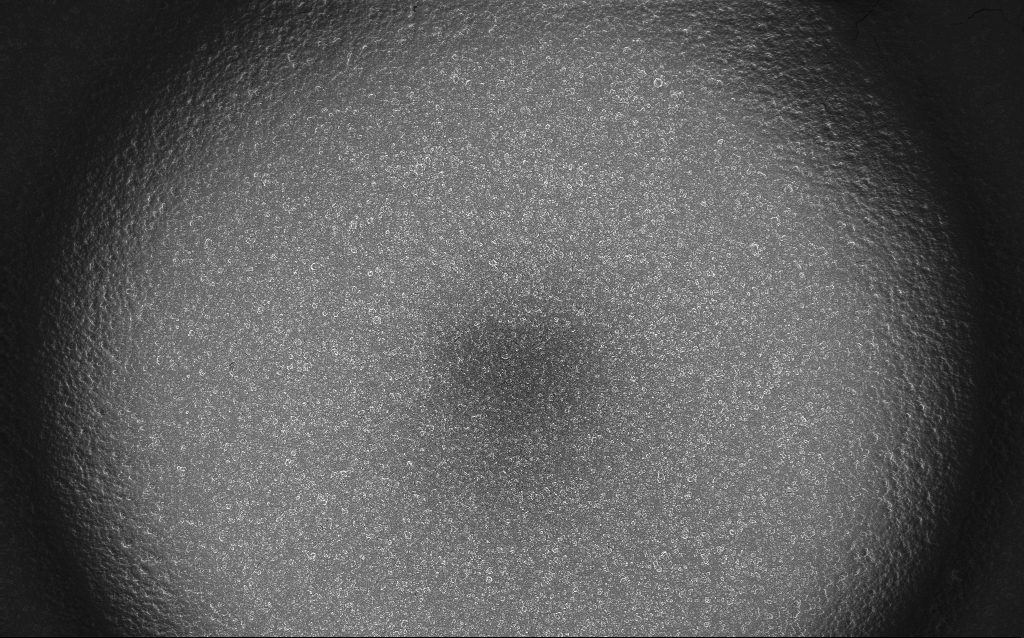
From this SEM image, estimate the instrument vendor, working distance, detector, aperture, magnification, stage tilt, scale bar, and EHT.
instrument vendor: Zeiss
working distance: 2.8 mm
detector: InLens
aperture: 30 µm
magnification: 0.122 K X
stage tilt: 0°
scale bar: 100000 nm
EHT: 10 kV